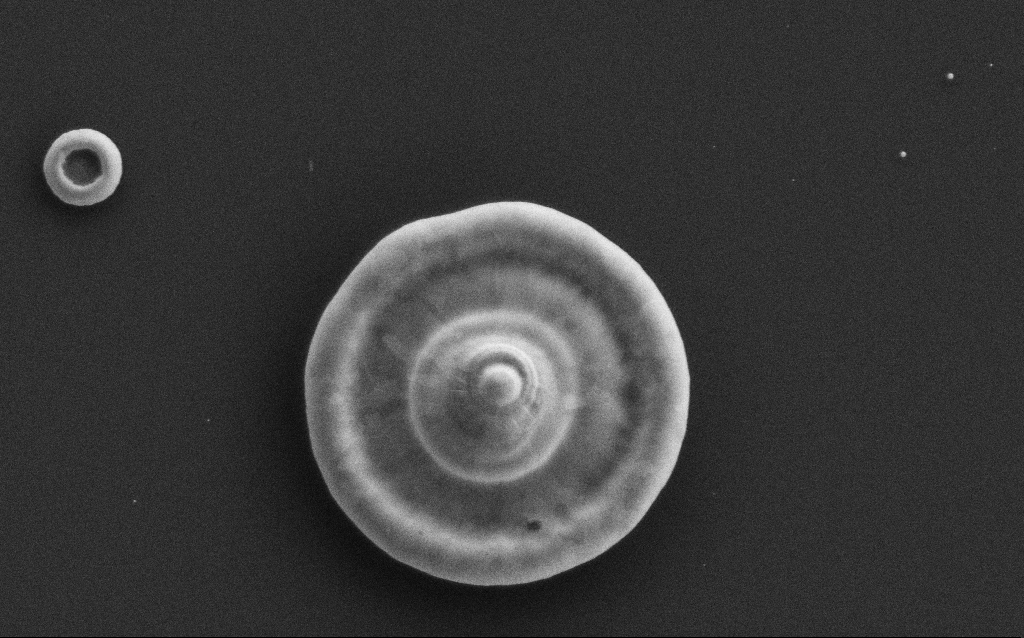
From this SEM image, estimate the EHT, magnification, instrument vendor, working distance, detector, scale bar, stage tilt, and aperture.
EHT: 3 kV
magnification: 30.26 K X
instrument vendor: Zeiss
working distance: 3 mm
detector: SE2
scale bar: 2000 nm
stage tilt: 0°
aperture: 30 µm